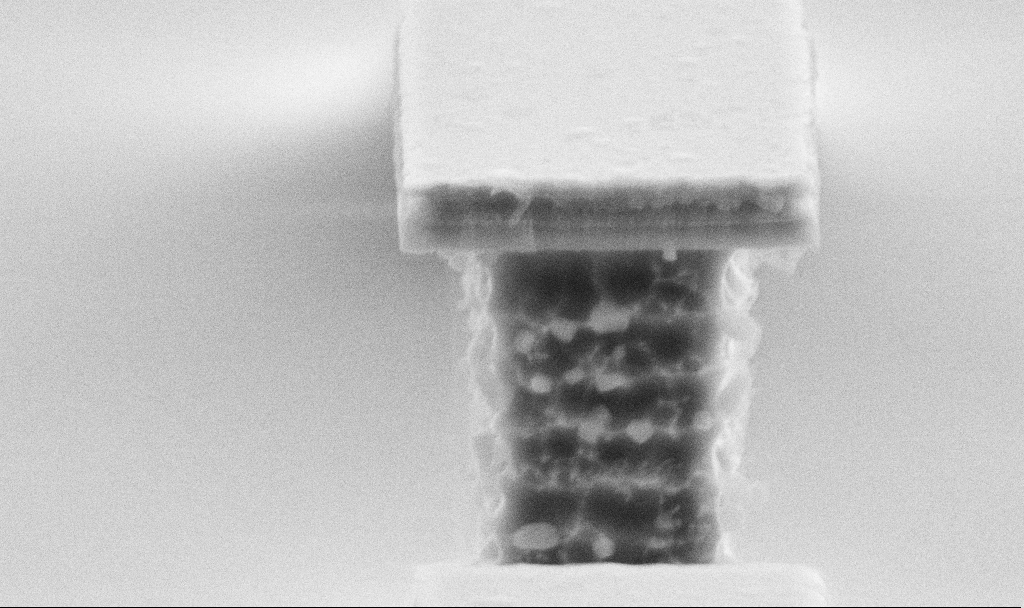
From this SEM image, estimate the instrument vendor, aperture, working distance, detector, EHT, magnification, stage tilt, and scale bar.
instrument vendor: Zeiss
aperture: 30 µm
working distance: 6.8 mm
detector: SE2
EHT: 5 kV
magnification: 51.91 K X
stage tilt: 70°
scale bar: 1000 nm